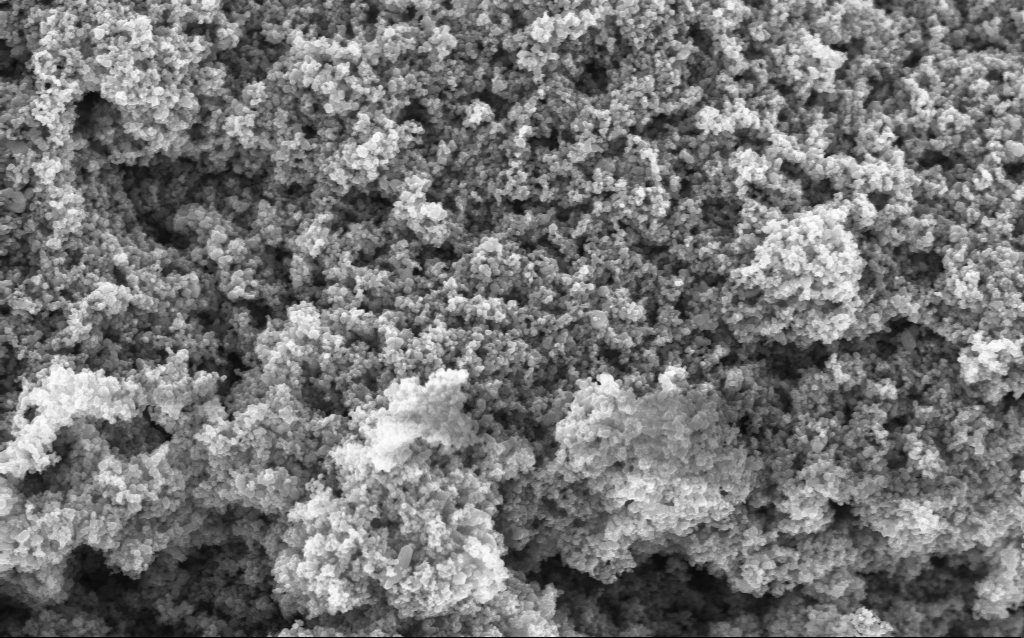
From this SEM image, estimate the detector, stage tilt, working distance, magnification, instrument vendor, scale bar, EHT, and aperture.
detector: InLens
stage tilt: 0°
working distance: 4.4 mm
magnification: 68.69 K X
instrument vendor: Zeiss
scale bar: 1000 nm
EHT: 5 kV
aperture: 30 µm